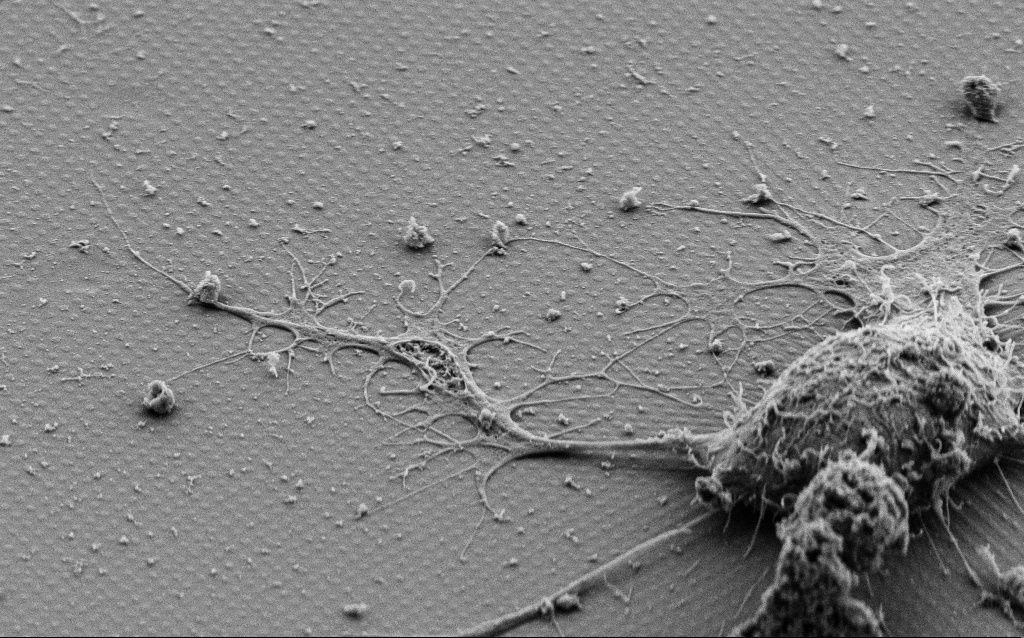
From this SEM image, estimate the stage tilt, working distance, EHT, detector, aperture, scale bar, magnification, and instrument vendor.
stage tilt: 45°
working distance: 6 mm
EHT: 3 kV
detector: SE2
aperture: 30 µm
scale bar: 2000 nm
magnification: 12.82 K X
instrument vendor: Zeiss